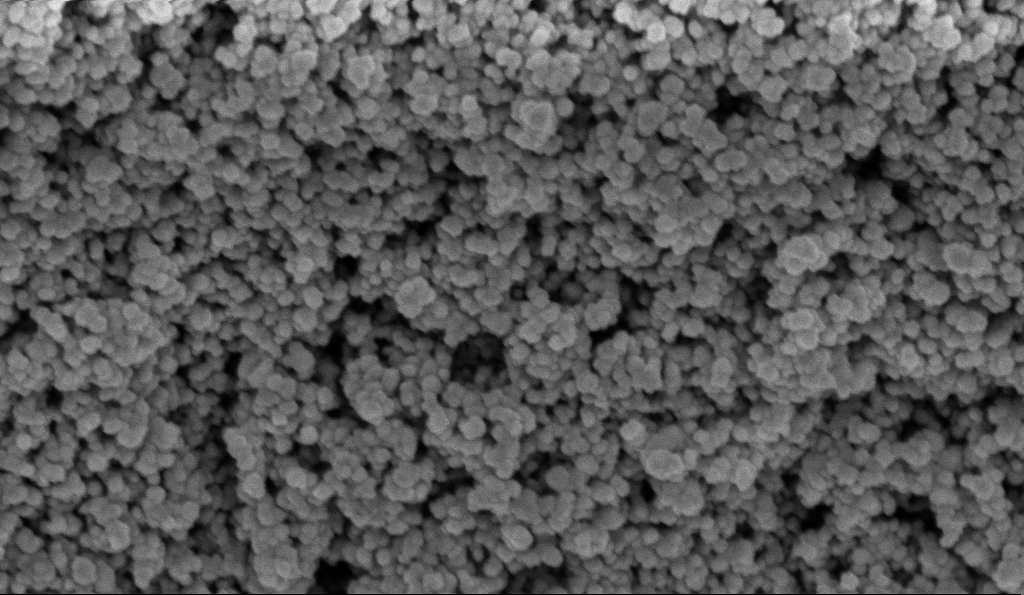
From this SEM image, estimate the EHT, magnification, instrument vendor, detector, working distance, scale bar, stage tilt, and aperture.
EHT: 5 kV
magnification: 135 K X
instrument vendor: Zeiss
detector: InLens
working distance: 5.9 mm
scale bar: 200 nm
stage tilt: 0°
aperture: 30 µm